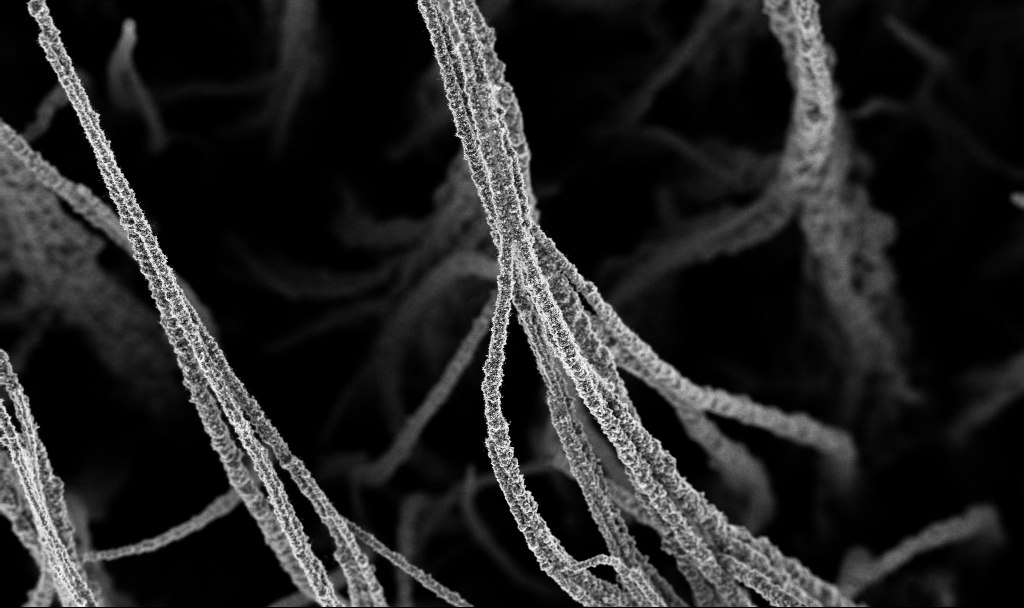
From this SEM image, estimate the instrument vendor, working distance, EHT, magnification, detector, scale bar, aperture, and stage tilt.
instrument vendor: Zeiss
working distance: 2.7 mm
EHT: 3 kV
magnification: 0.5 K X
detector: InLens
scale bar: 100000 nm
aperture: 30 µm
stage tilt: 0°